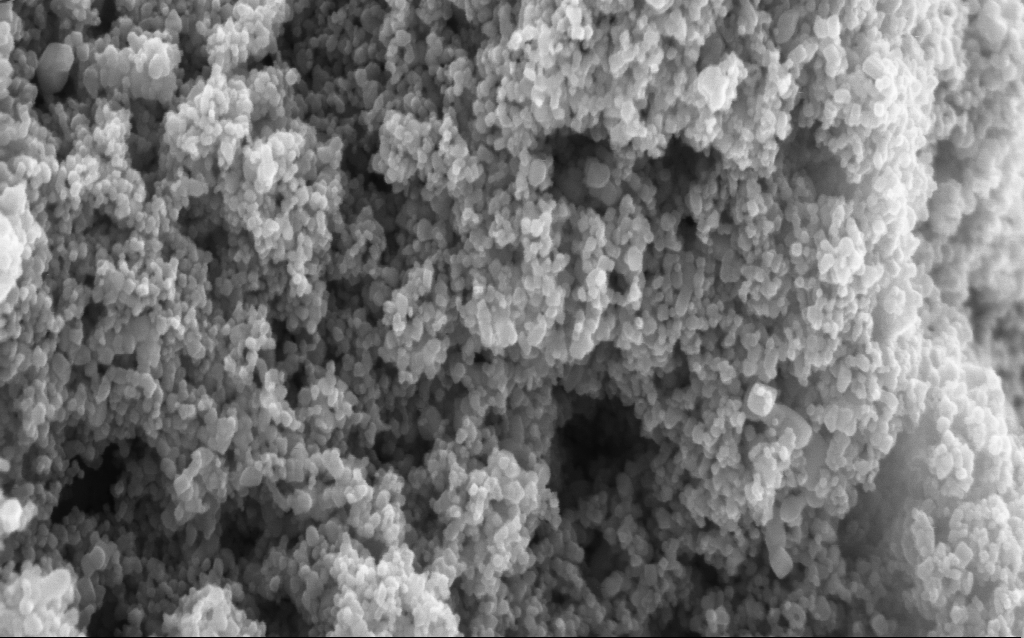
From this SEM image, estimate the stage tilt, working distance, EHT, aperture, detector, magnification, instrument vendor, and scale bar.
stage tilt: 0°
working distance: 4.7 mm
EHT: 5 kV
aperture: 30 µm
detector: InLens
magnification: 138.82 K X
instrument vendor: Zeiss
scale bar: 200 nm